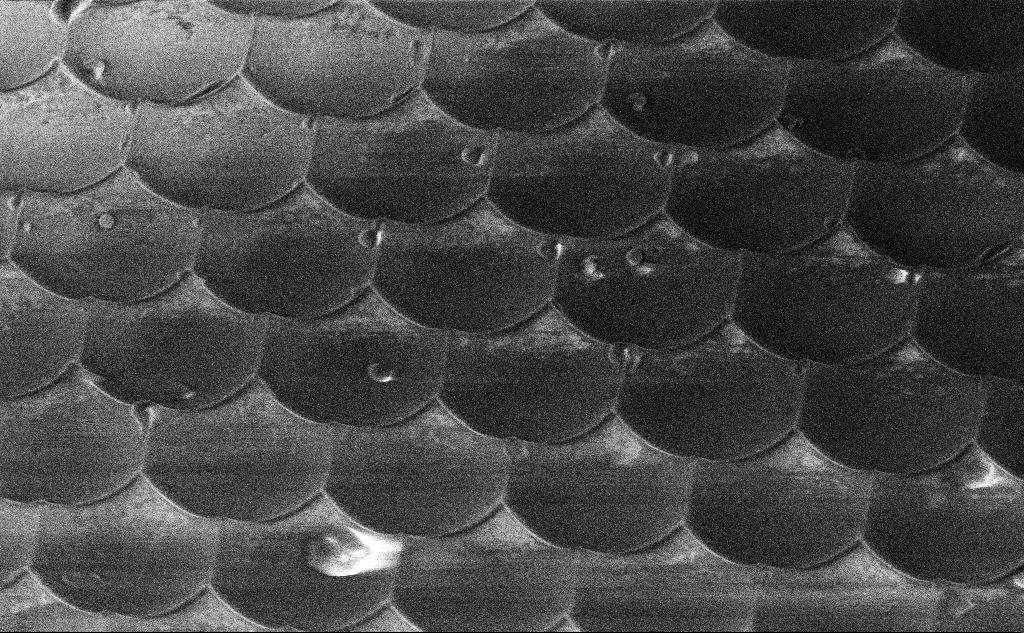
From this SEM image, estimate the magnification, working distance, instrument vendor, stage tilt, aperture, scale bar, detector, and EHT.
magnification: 22.04 K X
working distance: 10.7 mm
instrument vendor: Zeiss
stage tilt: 45°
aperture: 30 µm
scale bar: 2000 nm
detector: InLens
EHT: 3 kV